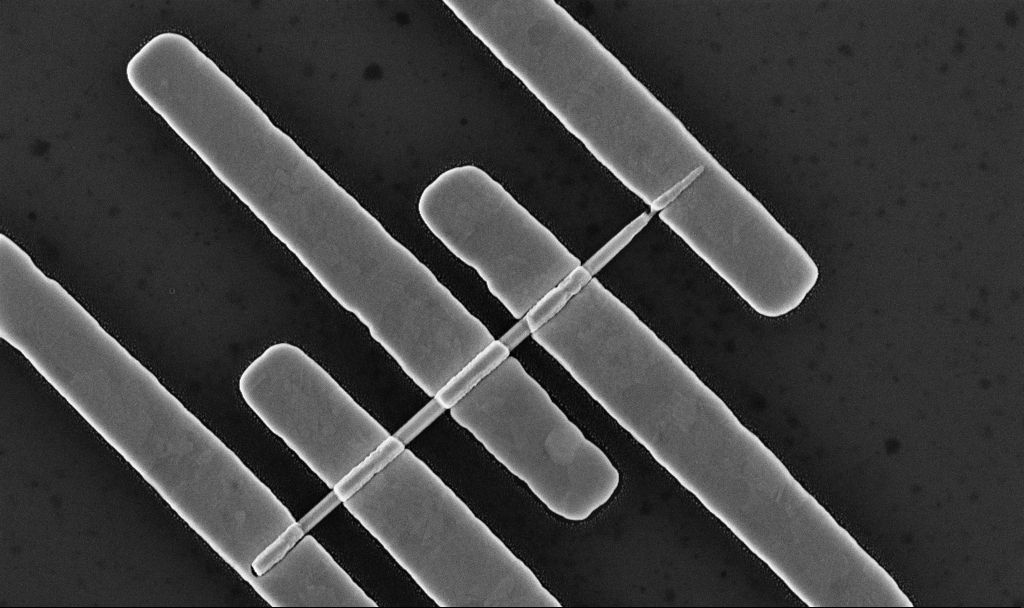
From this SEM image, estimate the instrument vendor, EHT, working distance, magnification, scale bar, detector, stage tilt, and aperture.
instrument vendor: Zeiss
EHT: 10 kV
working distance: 7.6 mm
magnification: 45.35 K X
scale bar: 1000 nm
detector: InLens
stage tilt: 0°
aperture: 30 µm